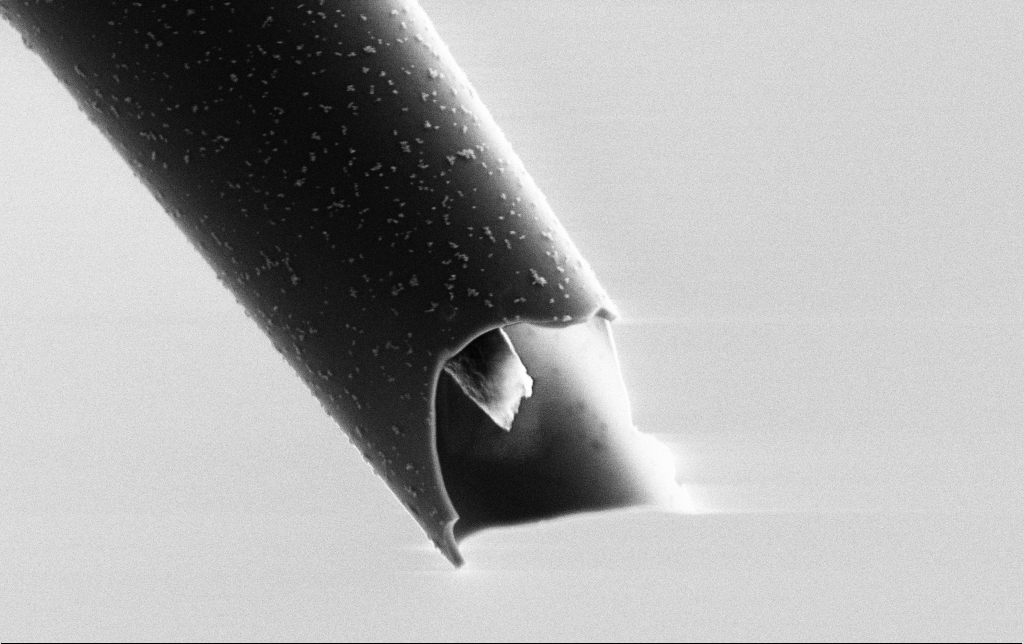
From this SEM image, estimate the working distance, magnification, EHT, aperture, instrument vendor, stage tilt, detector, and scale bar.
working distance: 6.7 mm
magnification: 25 K X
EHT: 3 kV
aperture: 30 µm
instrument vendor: Zeiss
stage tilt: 0°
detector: SE2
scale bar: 2000 nm